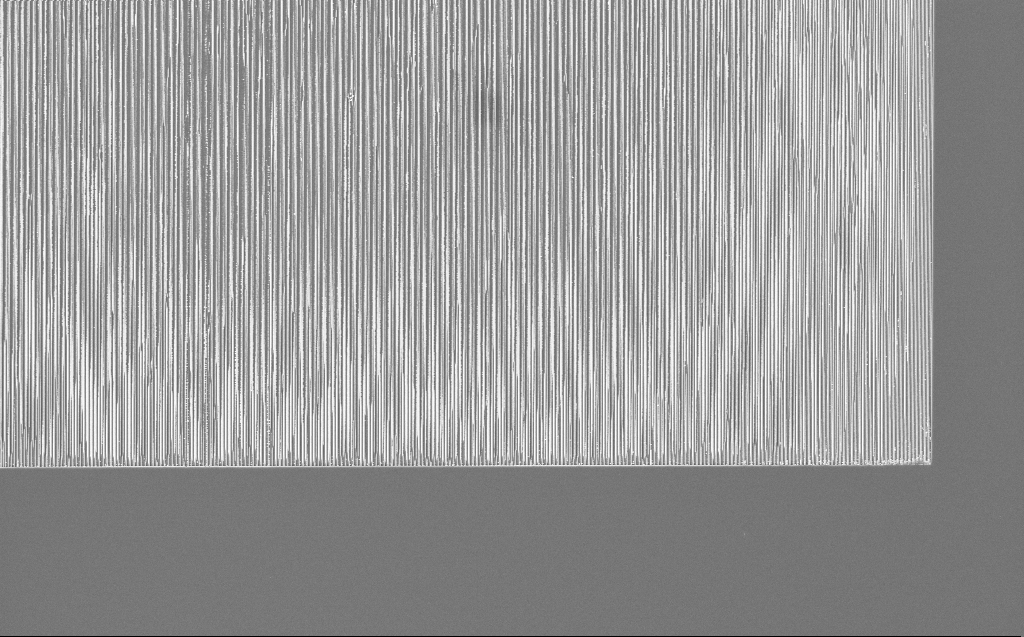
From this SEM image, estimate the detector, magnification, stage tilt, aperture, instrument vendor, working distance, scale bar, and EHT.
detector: InLens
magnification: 3.31 K X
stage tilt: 0°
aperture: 30 µm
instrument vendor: Zeiss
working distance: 7 mm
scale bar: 10000 nm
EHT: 5 kV